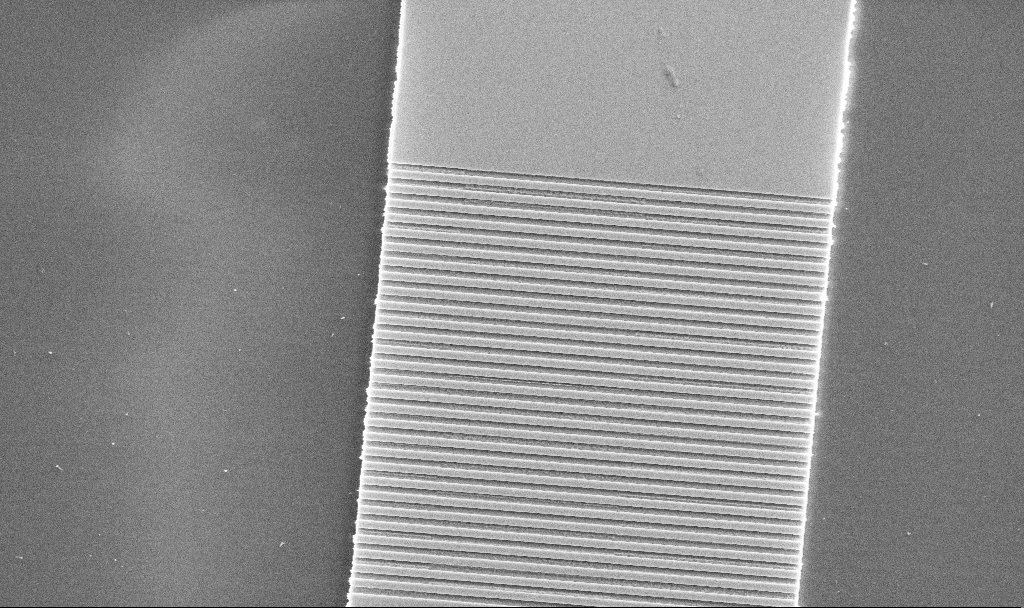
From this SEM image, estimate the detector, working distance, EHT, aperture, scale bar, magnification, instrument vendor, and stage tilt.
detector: SE2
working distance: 7.4 mm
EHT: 5 kV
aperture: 30 µm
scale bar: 2000 nm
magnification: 8.25 K X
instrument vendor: Zeiss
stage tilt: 0°